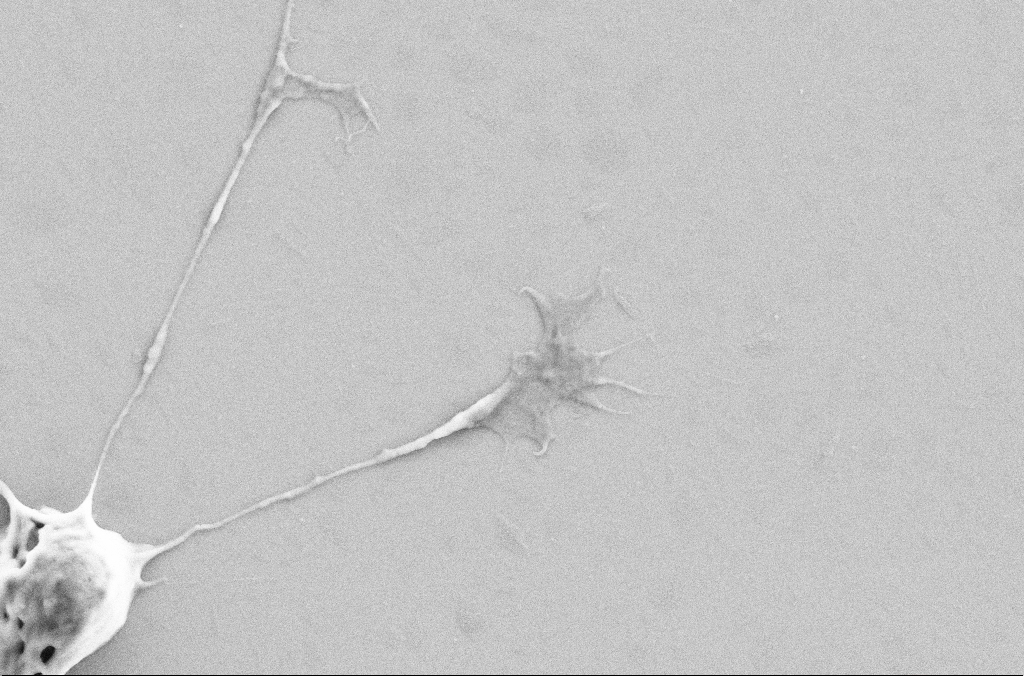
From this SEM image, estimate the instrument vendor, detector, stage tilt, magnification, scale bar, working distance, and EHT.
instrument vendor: Zeiss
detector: SE2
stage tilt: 0°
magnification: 10 K X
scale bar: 2000 nm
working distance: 3.2 mm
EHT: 5 kV